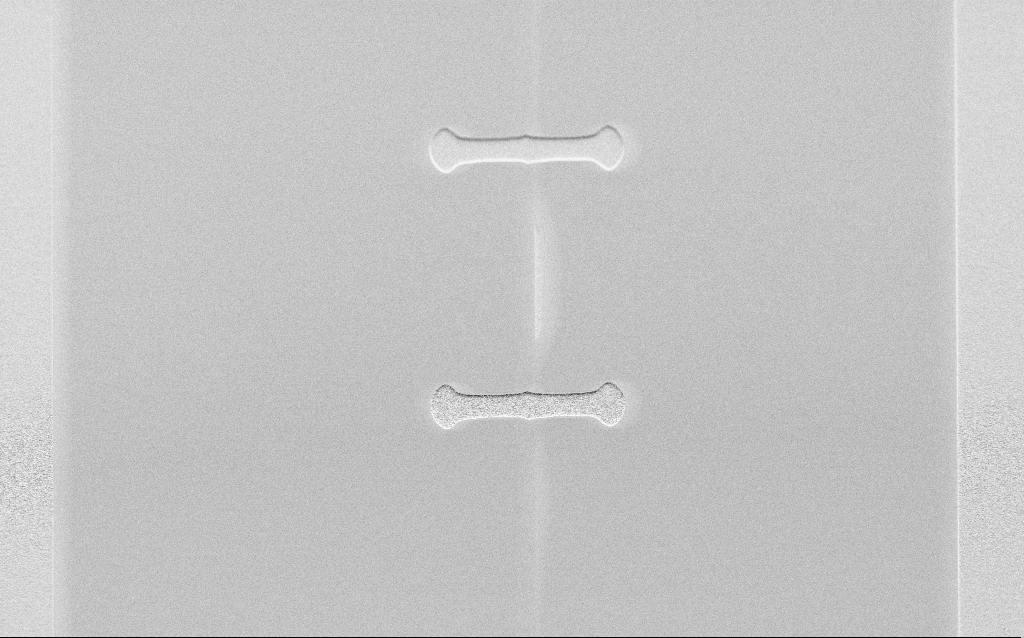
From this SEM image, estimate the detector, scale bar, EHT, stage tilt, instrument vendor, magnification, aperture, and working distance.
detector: SE2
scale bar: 20000 nm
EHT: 3 kV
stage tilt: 45°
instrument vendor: Zeiss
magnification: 0.957 K X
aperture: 30 µm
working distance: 8 mm